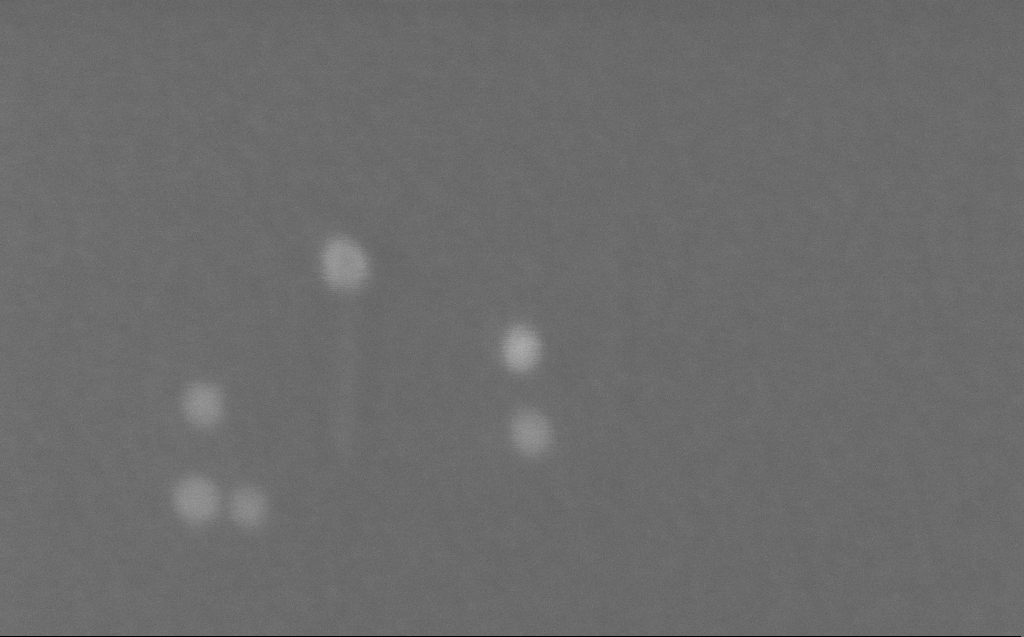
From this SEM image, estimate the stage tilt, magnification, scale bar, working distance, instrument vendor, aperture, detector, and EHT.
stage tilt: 0°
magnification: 1009.67 K X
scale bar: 20 nm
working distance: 3 mm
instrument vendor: Zeiss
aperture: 30 µm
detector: InLens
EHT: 10 kV